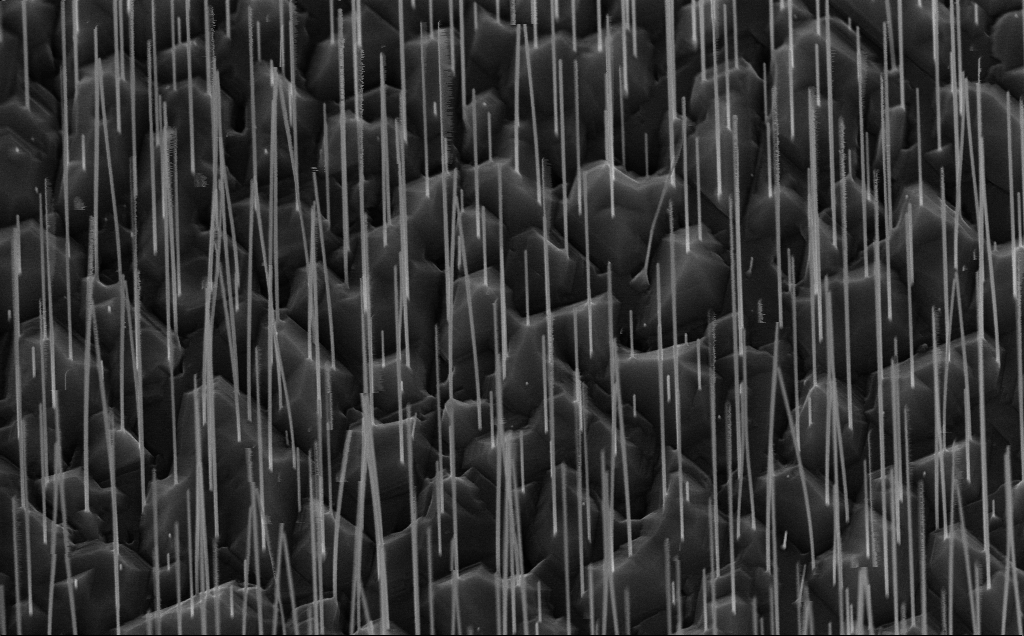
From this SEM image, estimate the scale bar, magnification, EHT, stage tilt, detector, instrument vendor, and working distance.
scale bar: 1000 nm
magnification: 37.95 K X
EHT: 10 kV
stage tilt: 44.9°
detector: InLens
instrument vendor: Zeiss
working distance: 7 mm